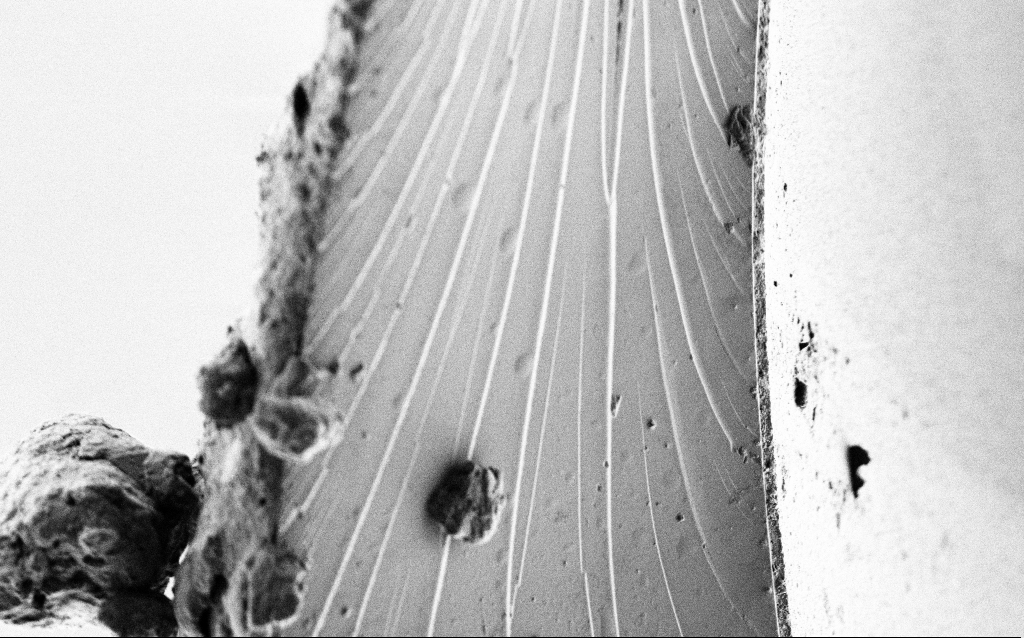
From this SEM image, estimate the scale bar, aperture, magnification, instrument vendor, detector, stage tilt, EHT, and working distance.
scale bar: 2000 nm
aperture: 30 µm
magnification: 10 K X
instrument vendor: Zeiss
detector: SE2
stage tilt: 45°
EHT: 1 kV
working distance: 5 mm